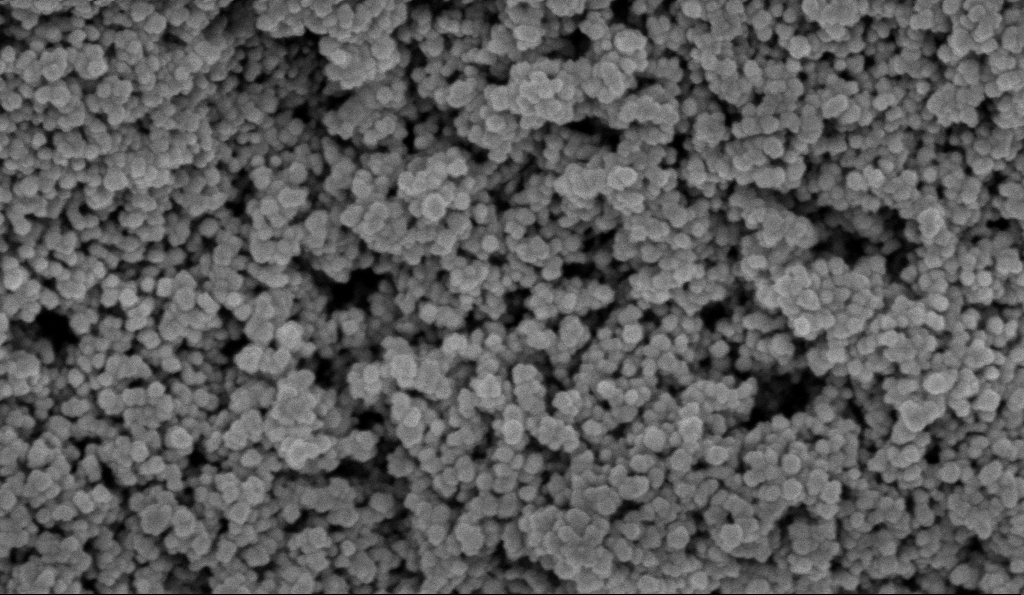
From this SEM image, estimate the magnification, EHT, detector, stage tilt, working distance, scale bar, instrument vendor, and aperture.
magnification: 135 K X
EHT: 5 kV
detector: InLens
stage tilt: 0°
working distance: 5.9 mm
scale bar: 200 nm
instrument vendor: Zeiss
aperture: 30 µm